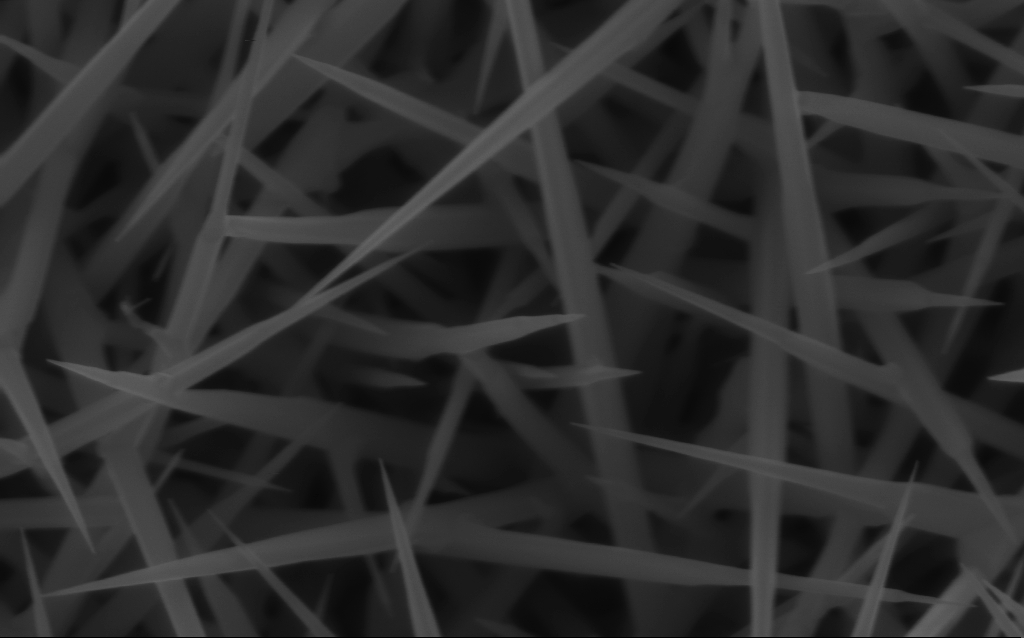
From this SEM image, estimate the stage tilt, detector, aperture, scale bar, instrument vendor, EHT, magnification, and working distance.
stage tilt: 0°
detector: InLens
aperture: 30 µm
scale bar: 200 nm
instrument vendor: Zeiss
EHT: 10 kV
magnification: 80 K X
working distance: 6 mm